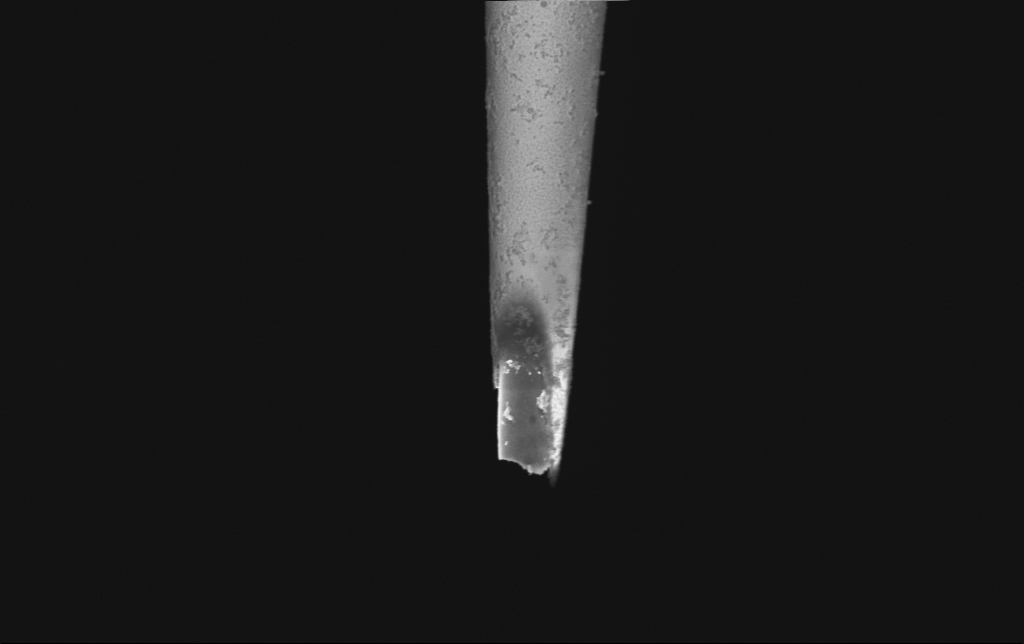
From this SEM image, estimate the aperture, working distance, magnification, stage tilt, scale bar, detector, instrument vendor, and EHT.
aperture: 30 µm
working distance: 6 mm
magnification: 15 K X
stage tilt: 0°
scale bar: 2000 nm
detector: InLens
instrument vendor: Zeiss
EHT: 2 kV